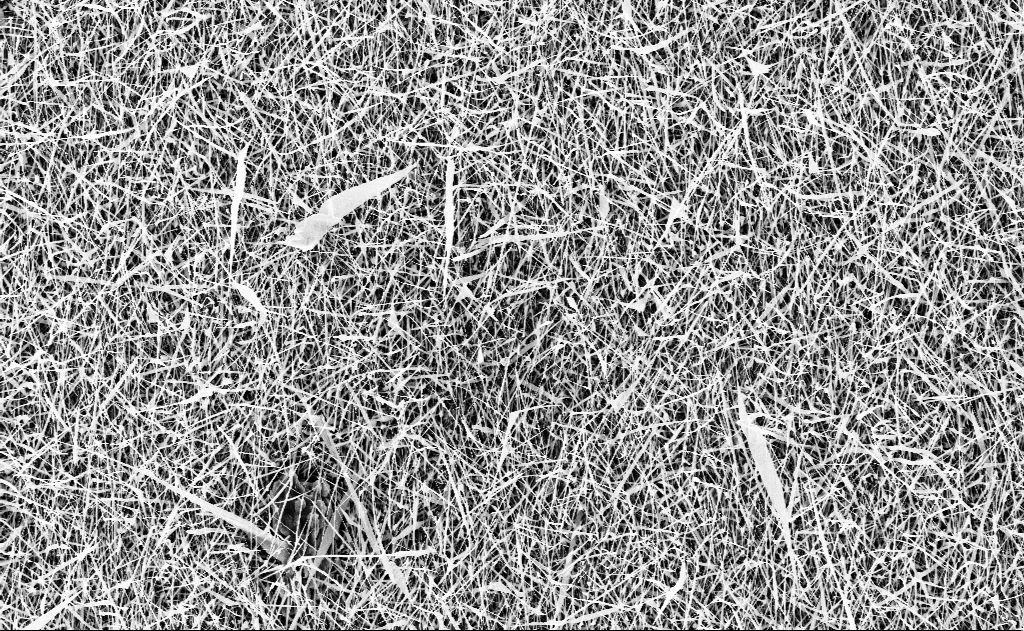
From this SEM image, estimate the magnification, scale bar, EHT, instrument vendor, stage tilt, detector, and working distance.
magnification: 5 K X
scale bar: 10000 nm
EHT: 10 kV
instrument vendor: Zeiss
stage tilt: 0°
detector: InLens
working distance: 16 mm